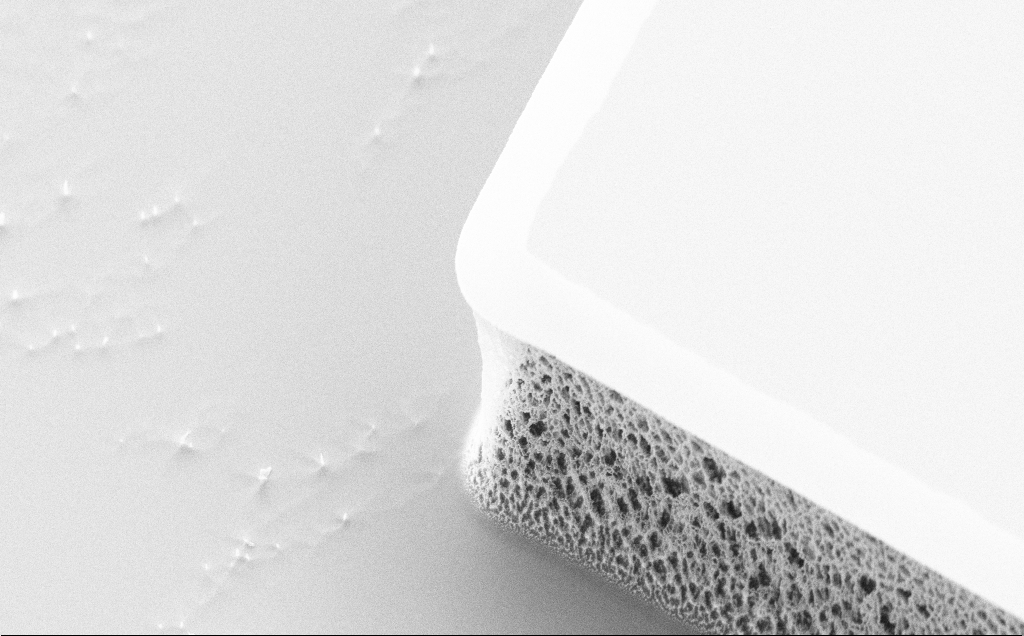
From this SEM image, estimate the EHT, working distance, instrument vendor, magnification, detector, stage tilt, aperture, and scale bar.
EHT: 5 kV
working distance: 8 mm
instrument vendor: Zeiss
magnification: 6.51 K X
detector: SE2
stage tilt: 45°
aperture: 30 µm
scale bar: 10000 nm